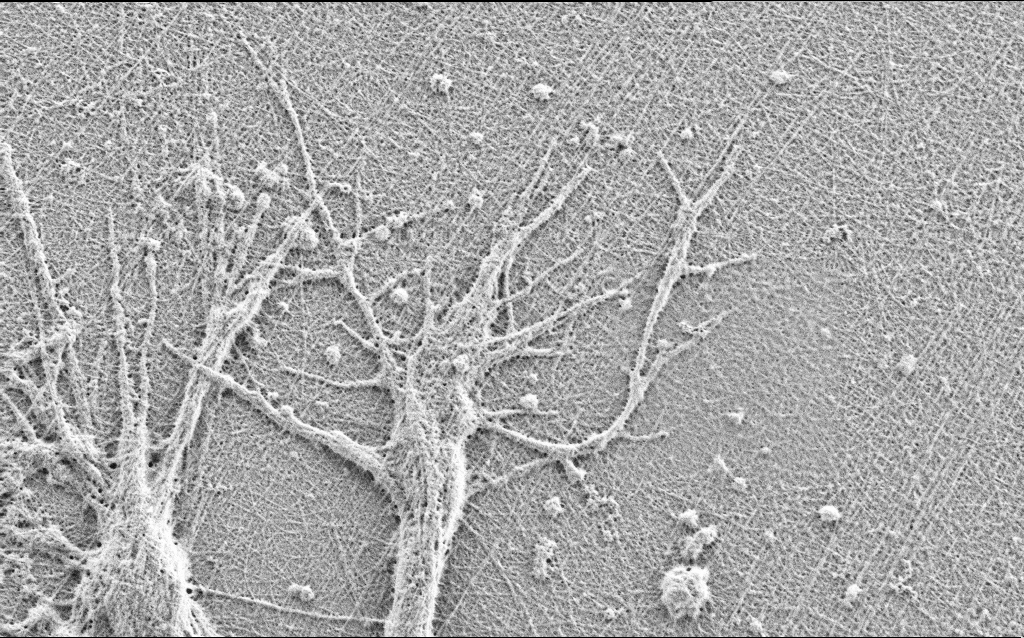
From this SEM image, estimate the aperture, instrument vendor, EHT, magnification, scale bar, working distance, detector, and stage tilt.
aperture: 30 µm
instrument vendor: Zeiss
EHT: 0.9 kV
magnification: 15 K X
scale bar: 2000 nm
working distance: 3 mm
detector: SE2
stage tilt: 0°